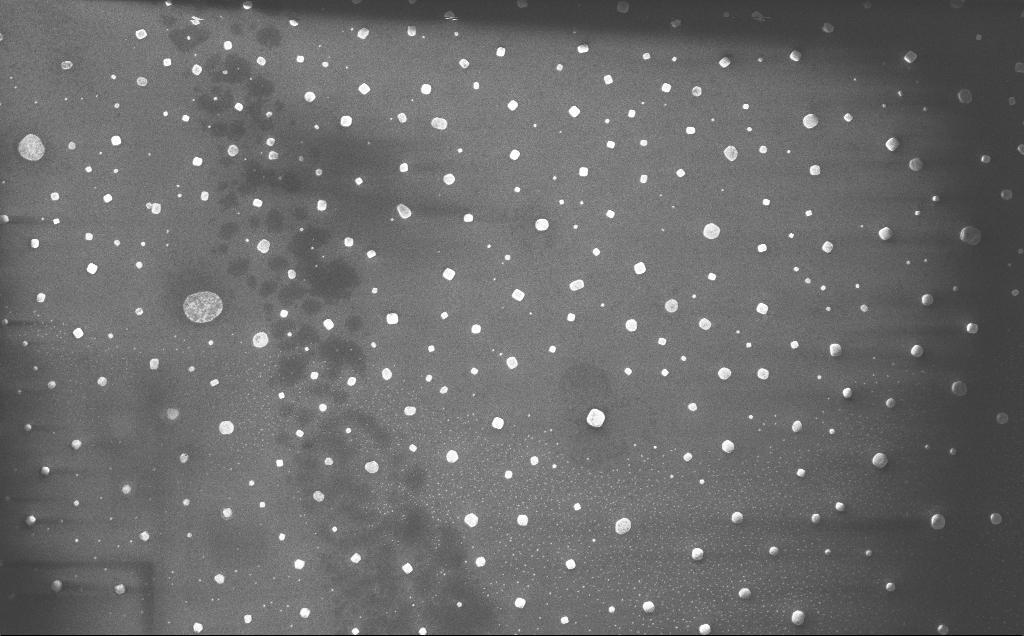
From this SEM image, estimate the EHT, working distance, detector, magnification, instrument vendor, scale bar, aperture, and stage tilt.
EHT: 10 kV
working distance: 4 mm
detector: InLens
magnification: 12.53 K X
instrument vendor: Zeiss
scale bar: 1000 nm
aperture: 30 µm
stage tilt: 0°